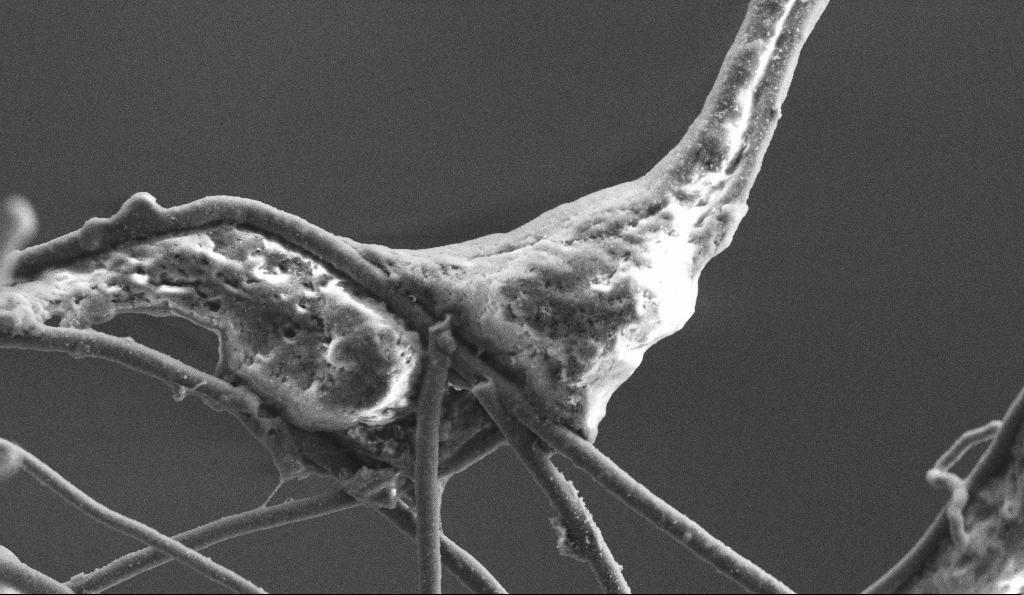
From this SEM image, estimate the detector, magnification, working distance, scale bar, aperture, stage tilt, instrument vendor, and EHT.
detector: SE2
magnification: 15 K X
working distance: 5.9 mm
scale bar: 2000 nm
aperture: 30 µm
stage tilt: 0°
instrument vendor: Zeiss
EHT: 3 kV